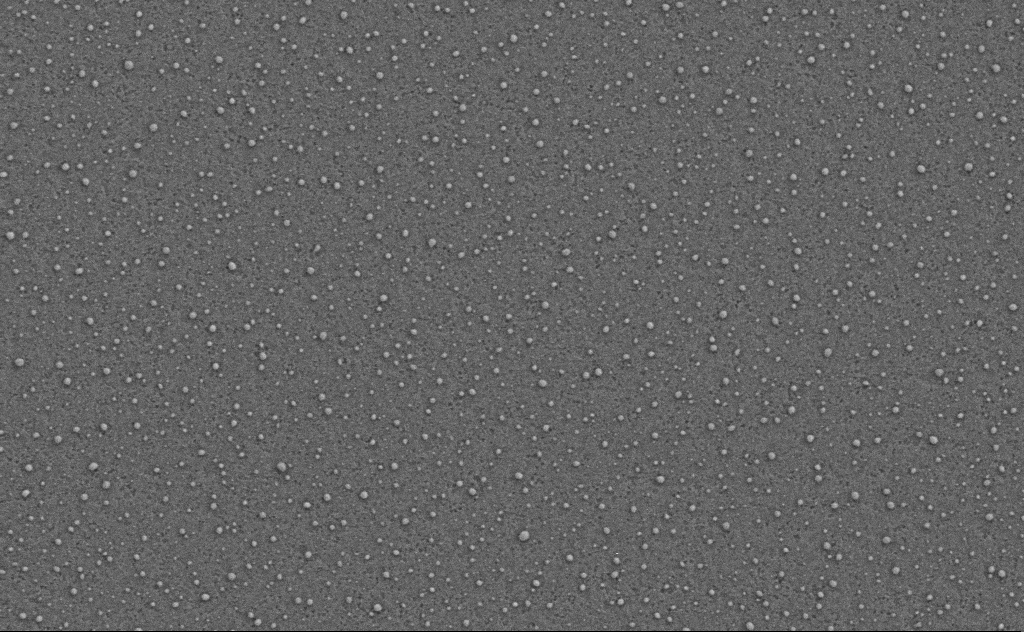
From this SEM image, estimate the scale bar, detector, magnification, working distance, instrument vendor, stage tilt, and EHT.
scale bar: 1000 nm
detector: SE2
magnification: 40 K X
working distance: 4 mm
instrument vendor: Zeiss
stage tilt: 0°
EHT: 3 kV